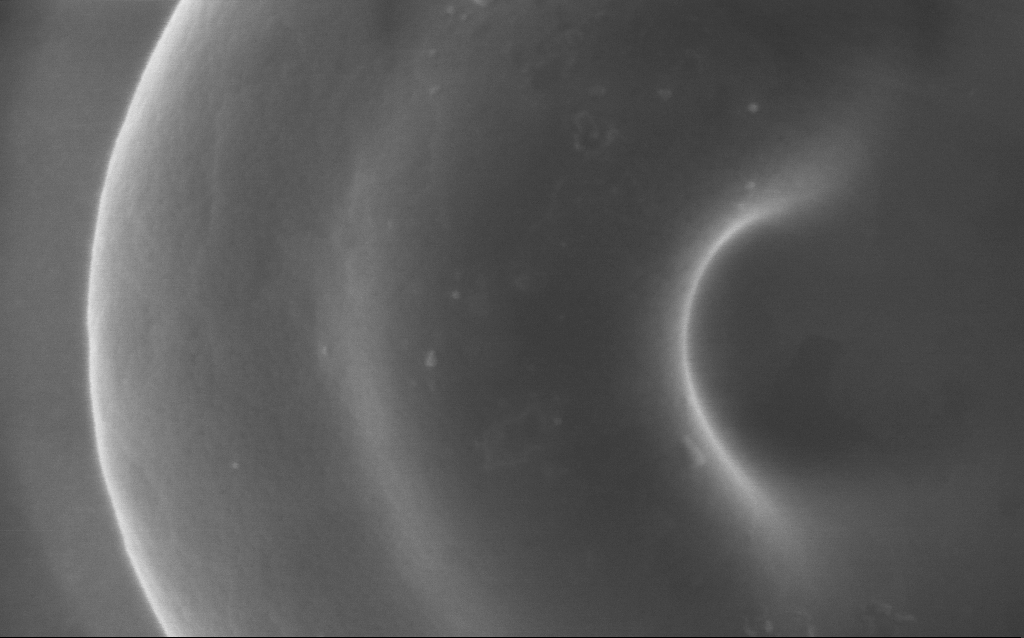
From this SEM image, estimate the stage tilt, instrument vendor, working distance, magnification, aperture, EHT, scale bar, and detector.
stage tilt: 0°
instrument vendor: Zeiss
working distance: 3 mm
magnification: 136 K X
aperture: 30 µm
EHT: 5 kV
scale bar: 200 nm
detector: InLens